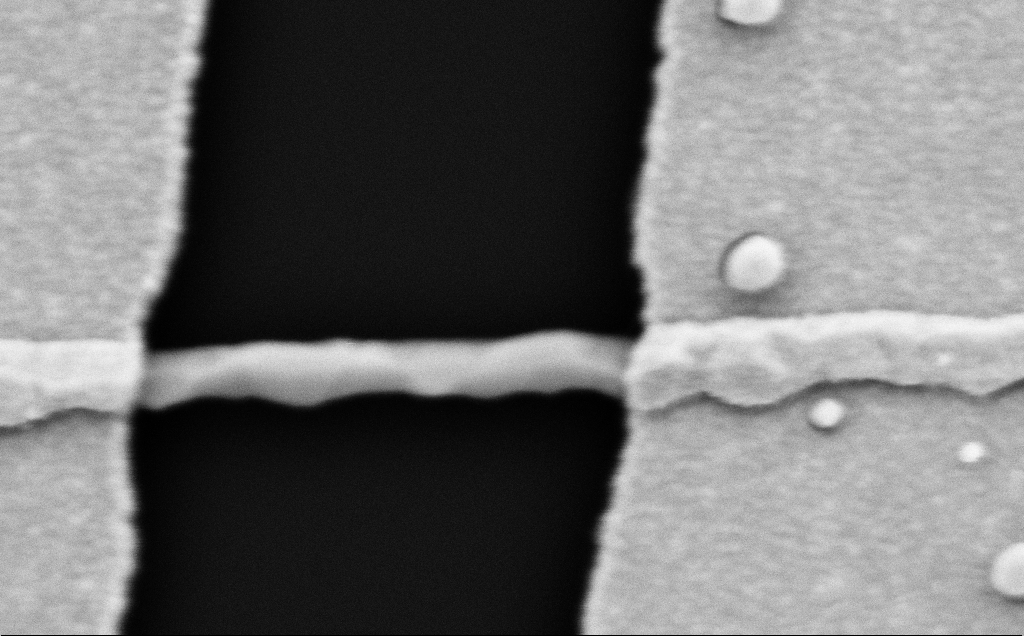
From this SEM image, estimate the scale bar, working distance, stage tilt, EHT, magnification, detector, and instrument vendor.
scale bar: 200 nm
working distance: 10 mm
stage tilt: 0°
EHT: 5 kV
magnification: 116.5 K X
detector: SE2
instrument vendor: Zeiss